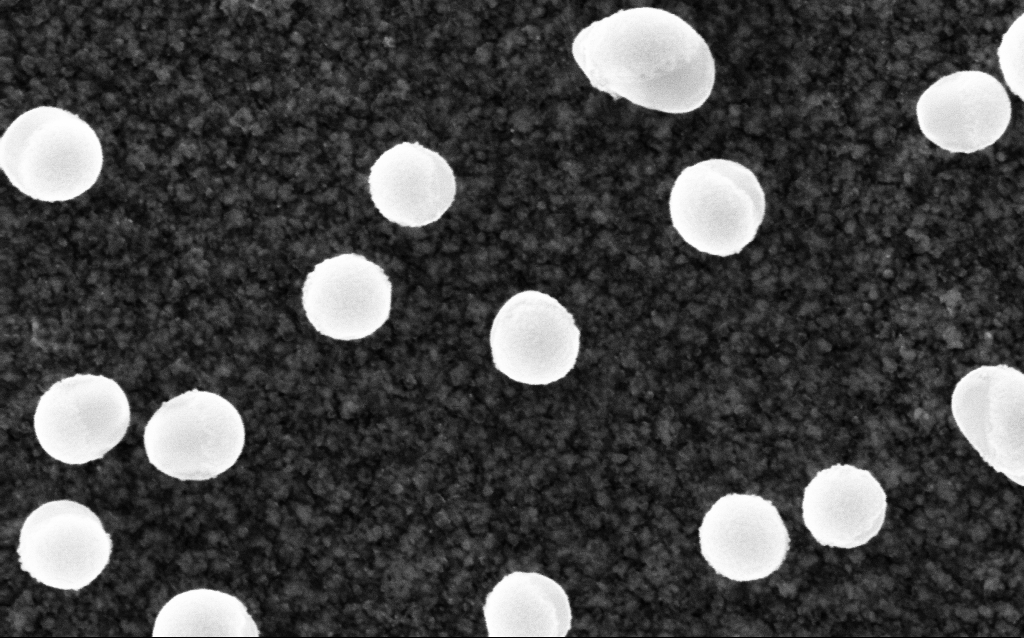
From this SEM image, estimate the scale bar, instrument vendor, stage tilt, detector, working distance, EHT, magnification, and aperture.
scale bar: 100 nm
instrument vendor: Zeiss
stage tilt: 0°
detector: InLens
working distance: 2.8 mm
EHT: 5 kV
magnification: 200 K X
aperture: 30 µm